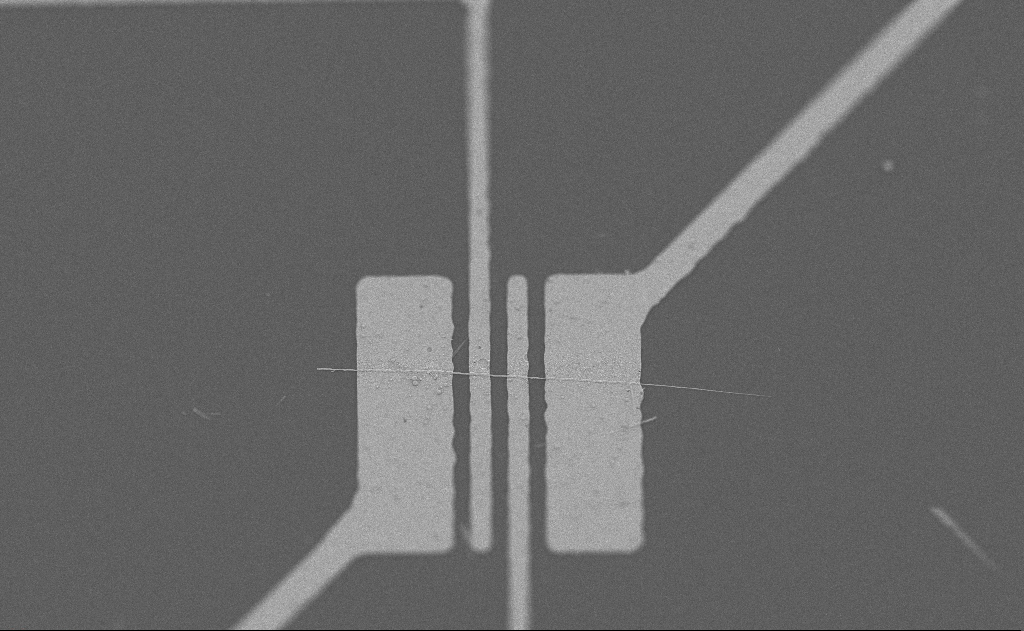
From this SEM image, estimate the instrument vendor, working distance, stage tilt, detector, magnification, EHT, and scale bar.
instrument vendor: Zeiss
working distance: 10 mm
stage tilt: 0°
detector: SE2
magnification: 3.47 K X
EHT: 5 kV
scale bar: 20000 nm